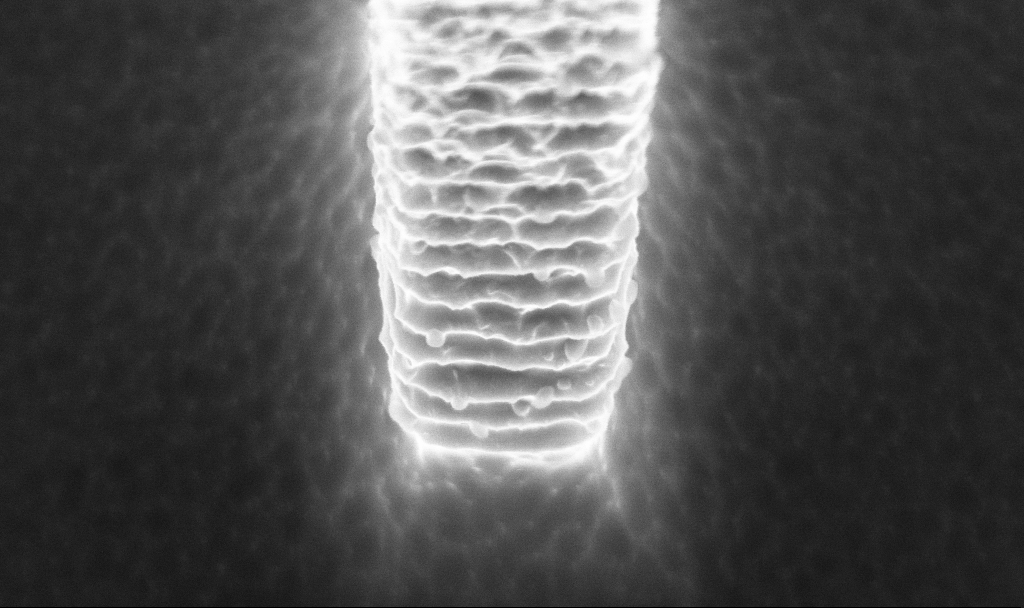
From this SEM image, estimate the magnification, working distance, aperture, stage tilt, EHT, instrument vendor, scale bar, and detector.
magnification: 68.03 K X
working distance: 4.5 mm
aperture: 30 µm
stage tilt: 20°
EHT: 5 kV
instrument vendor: Zeiss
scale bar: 1000 nm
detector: InLens